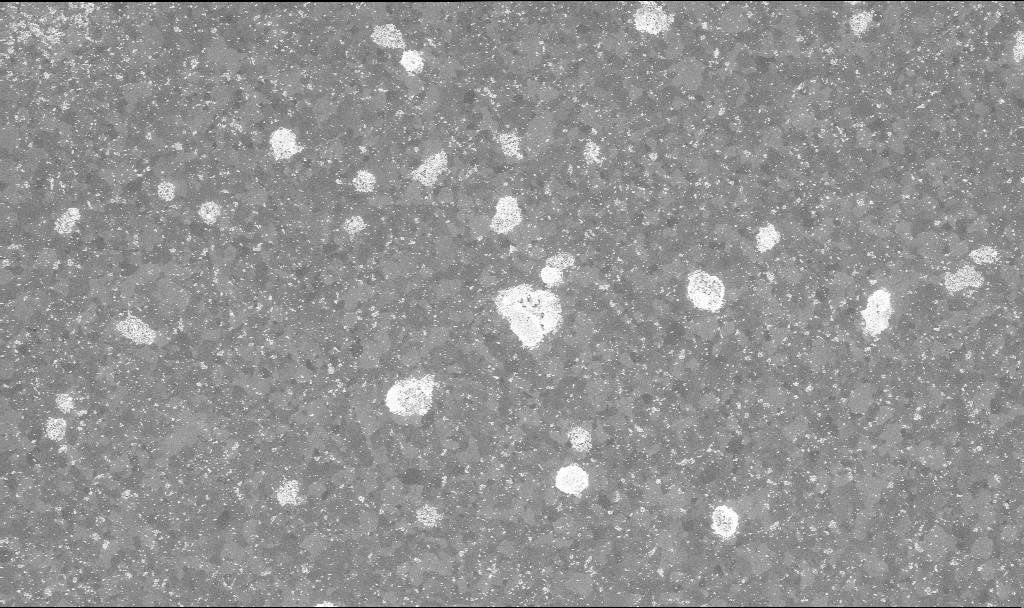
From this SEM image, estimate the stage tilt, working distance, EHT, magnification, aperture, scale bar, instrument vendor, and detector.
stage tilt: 0°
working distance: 3.8 mm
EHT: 10 kV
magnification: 20 K X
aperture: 30 µm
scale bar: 1000 nm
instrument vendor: Zeiss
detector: InLens